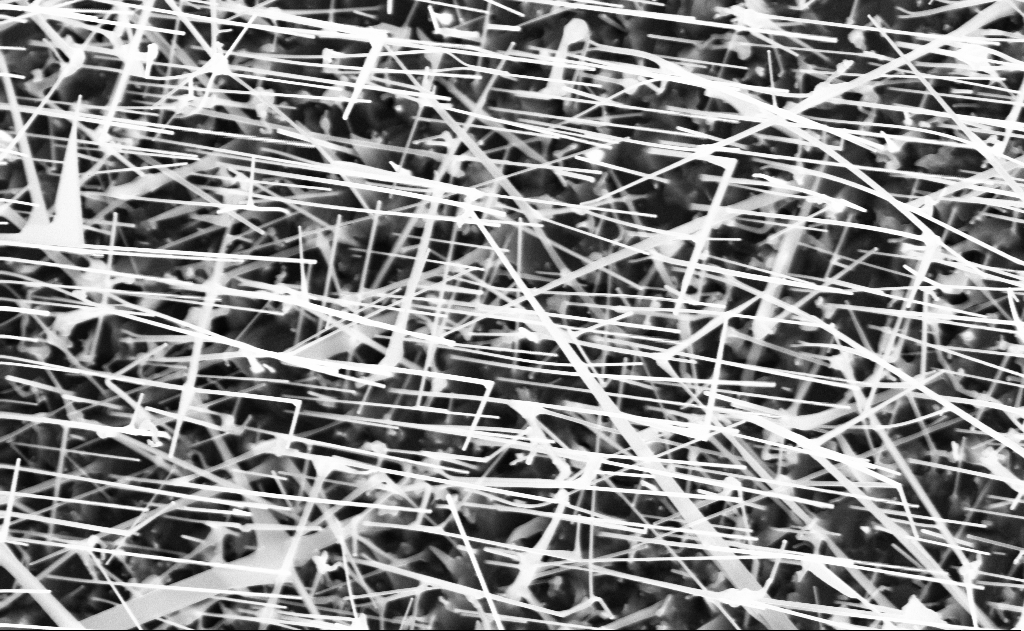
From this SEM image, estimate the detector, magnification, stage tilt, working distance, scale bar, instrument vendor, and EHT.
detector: InLens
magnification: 40 K X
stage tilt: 0°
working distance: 10 mm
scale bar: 1000 nm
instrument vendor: Zeiss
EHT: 10 kV